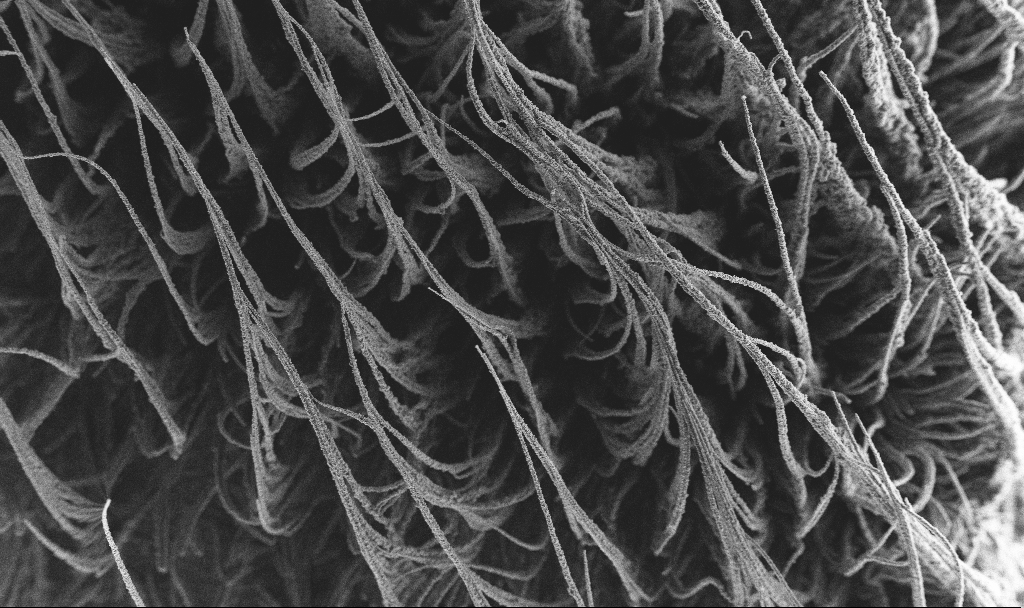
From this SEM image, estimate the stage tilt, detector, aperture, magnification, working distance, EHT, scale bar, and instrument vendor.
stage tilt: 0°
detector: SE2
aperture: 30 µm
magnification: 0.15 K X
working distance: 2.9 mm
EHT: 3 kV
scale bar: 100000 nm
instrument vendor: Zeiss